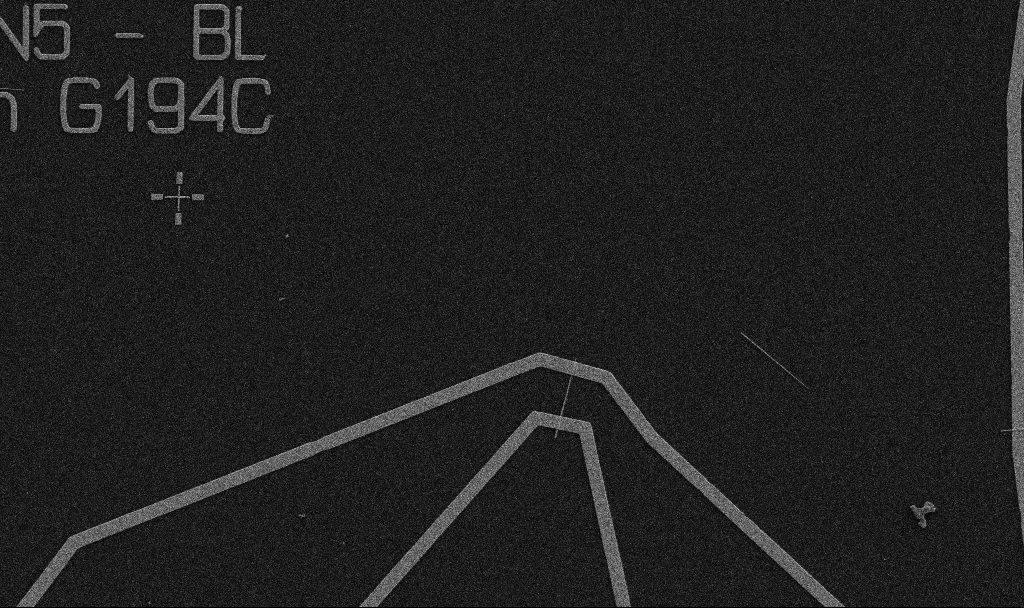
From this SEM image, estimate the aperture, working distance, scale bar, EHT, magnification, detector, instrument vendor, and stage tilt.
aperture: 30 µm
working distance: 10.7 mm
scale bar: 10000 nm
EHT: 5 kV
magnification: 5 K X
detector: SE2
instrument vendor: Zeiss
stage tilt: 0°